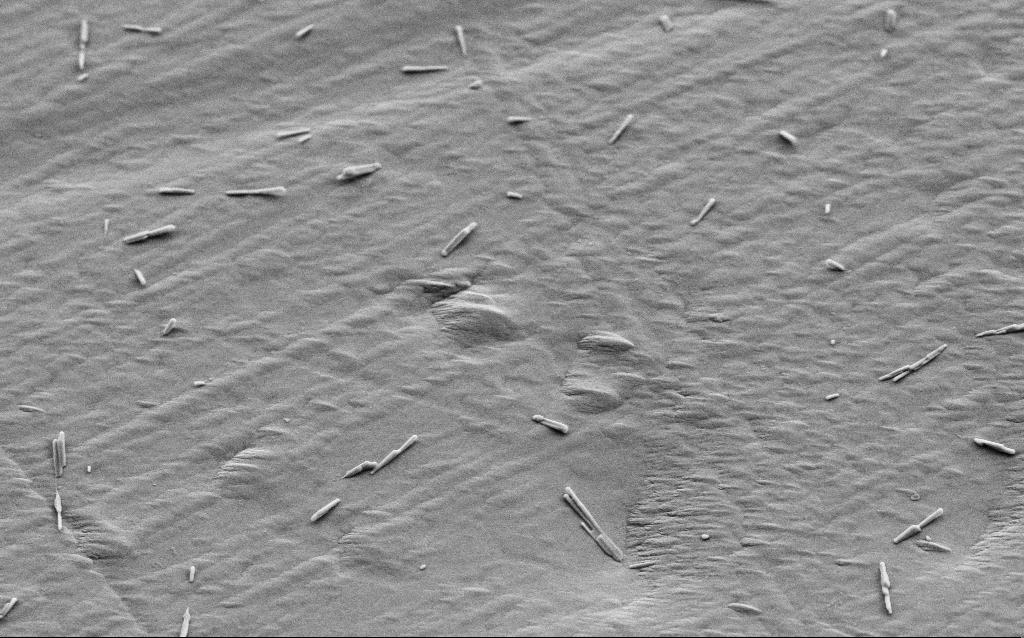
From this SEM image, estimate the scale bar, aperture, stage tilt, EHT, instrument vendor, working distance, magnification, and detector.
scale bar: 2000 nm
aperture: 30 µm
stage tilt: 35°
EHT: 5 kV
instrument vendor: Zeiss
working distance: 4 mm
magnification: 13.45 K X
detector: SE2